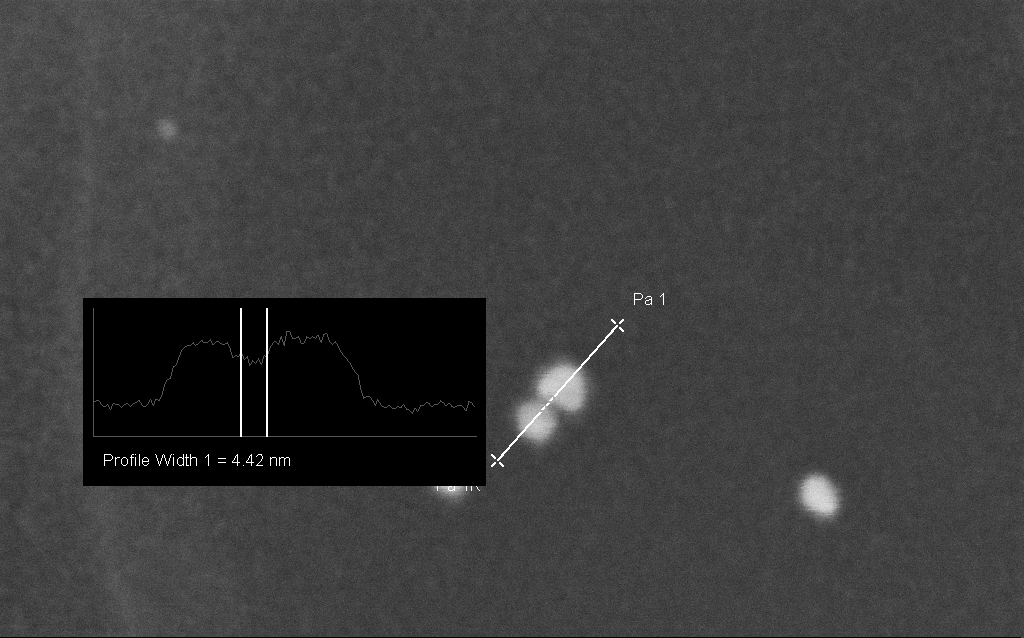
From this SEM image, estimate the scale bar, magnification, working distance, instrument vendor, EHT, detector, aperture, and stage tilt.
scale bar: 20 nm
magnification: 1000 K X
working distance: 2.1 mm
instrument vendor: Zeiss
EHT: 10 kV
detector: InLens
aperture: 30 µm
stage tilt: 0°